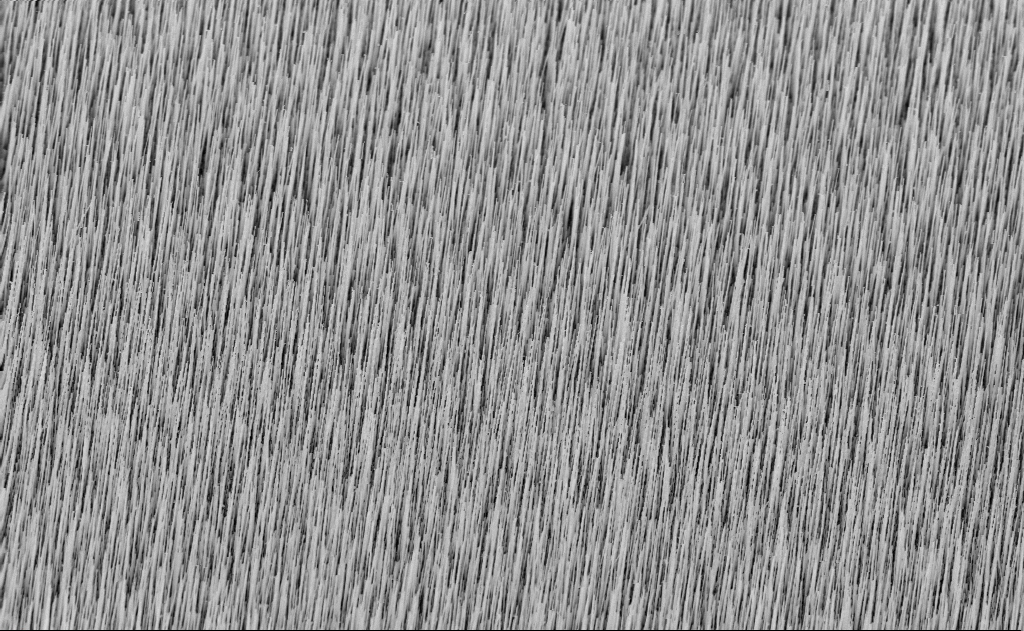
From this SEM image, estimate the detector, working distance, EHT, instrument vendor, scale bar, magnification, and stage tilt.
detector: InLens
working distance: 9 mm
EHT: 10 kV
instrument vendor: Zeiss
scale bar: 10000 nm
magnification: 5 K X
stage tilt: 30°